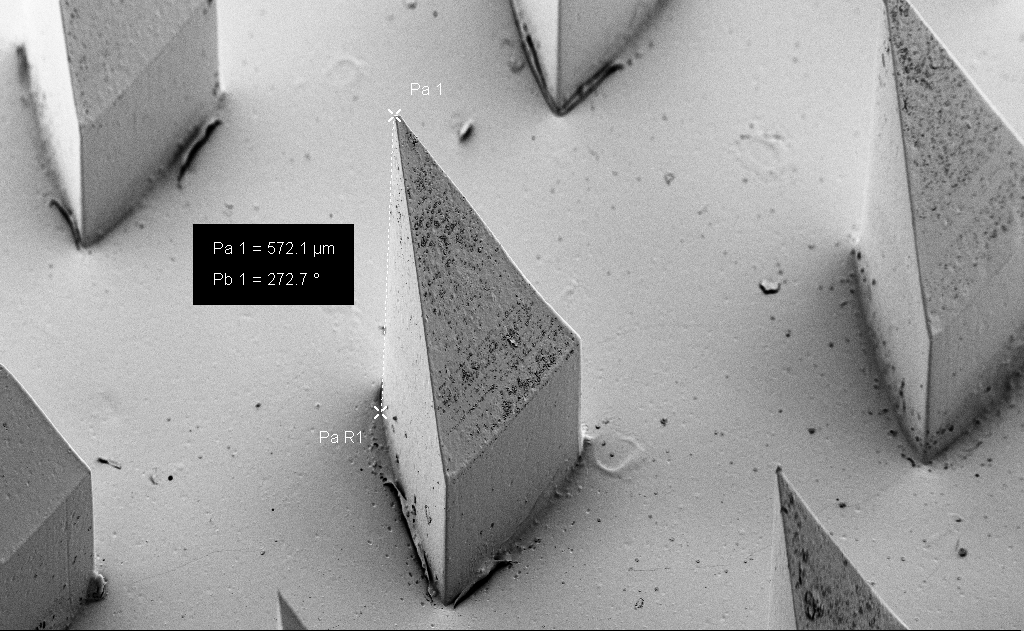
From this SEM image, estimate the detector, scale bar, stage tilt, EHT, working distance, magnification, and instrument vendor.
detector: SE2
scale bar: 100000 nm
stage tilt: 45°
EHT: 5 kV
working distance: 9 mm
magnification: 0.191 K X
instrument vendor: Zeiss